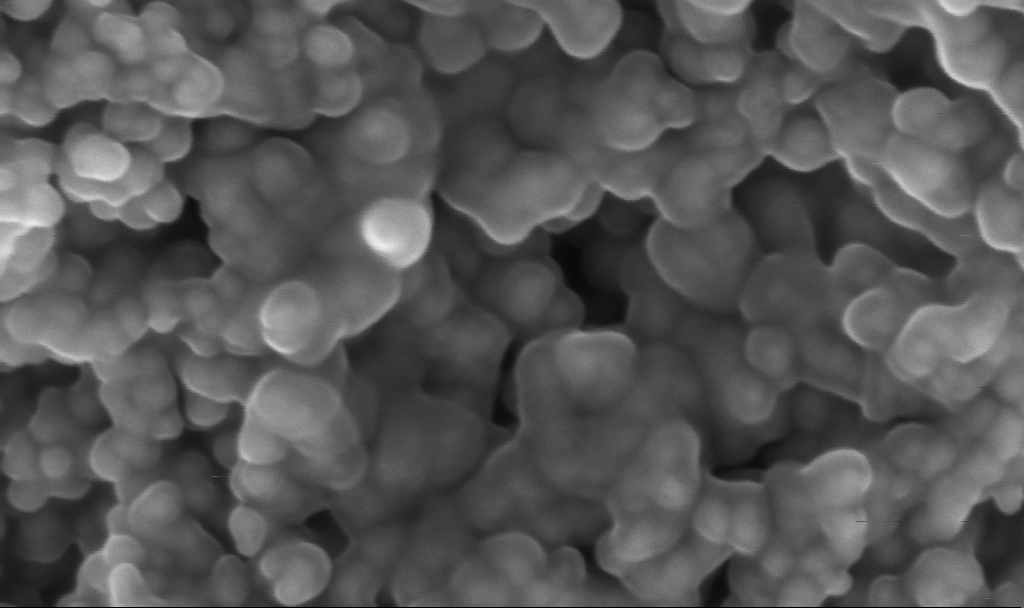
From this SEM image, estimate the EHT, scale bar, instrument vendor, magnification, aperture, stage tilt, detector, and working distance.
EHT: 3 kV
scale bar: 100 nm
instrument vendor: Zeiss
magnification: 350 K X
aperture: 30 µm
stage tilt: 0°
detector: InLens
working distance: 2.4 mm